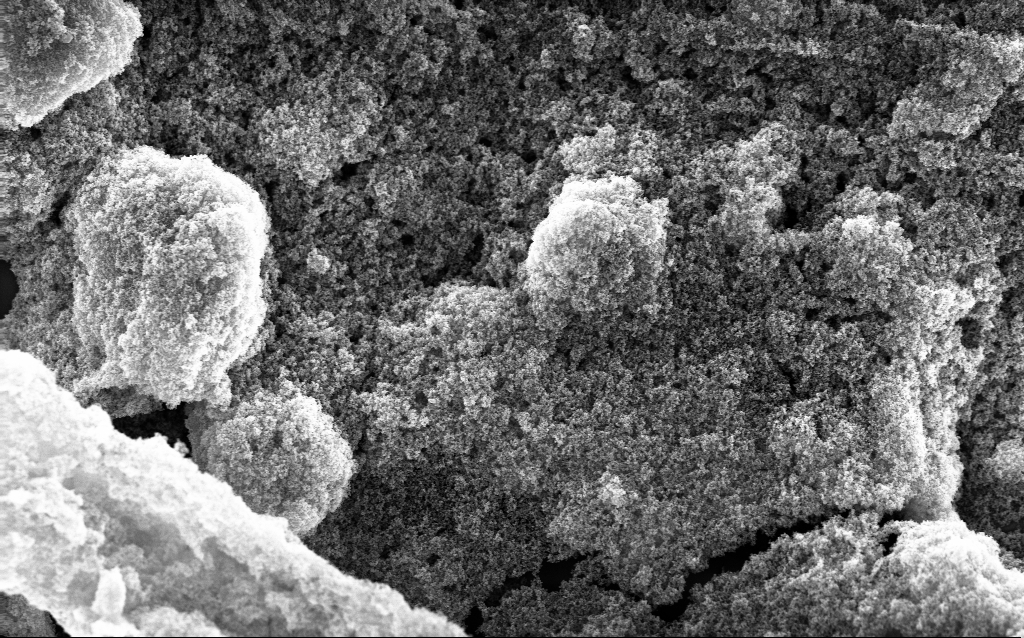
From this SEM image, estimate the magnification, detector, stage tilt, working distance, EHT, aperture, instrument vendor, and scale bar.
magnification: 20.87 K X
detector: InLens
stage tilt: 0°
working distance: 2.7 mm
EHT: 10 kV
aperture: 30 µm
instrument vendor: Zeiss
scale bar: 1000 nm